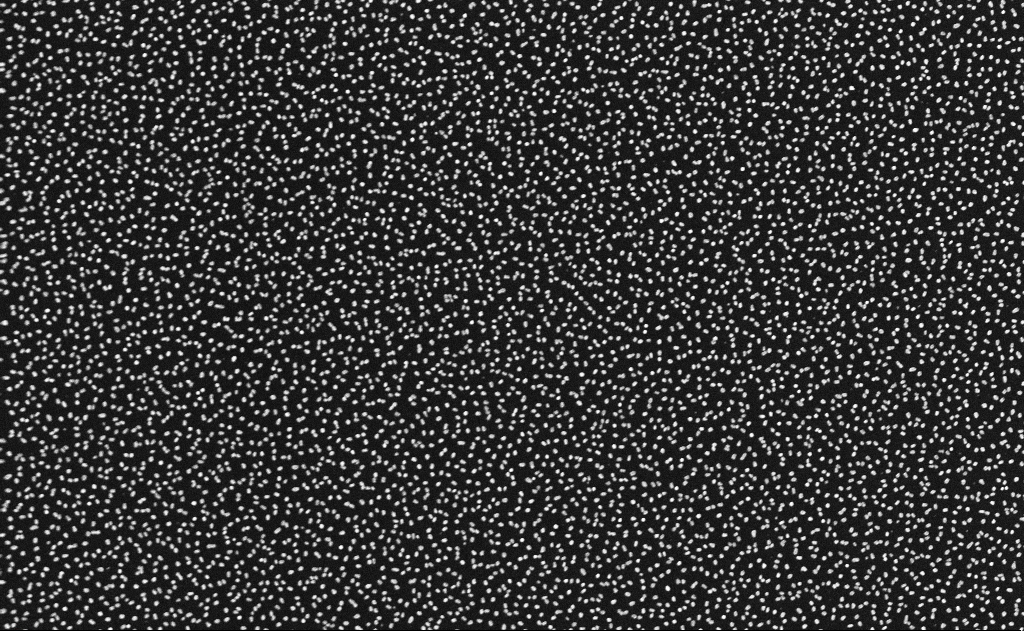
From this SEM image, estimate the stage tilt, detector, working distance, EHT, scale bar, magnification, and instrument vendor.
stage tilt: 0°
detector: InLens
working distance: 11 mm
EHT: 10 kV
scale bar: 2000 nm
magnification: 20 K X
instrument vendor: Zeiss